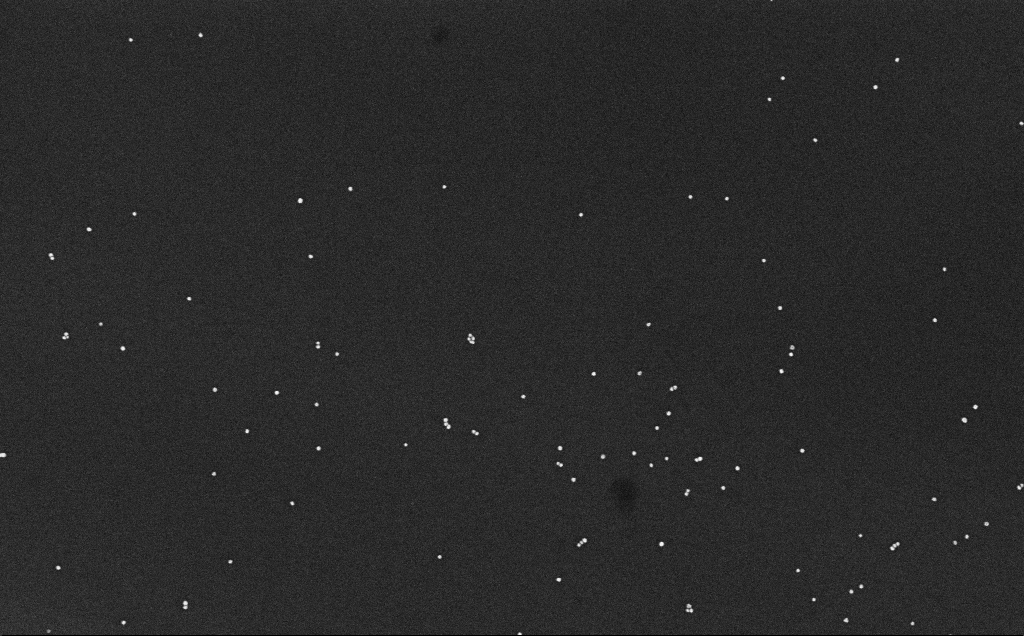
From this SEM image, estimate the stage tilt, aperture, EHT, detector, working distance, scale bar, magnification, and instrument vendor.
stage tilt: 0°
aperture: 30 µm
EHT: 10 kV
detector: InLens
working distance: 6.6 mm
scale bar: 200 nm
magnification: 100 K X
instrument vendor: Zeiss